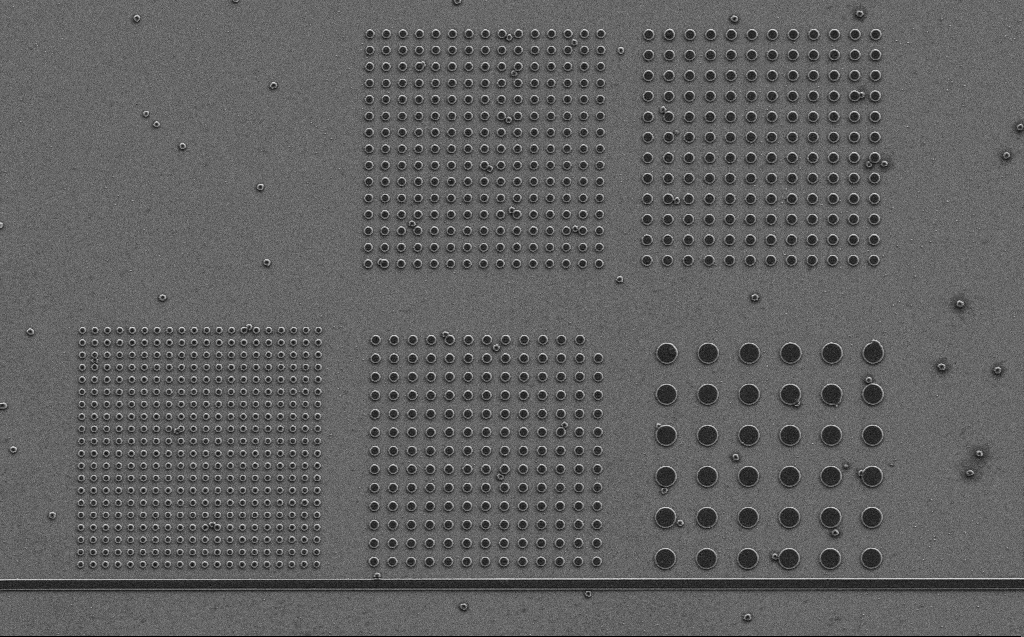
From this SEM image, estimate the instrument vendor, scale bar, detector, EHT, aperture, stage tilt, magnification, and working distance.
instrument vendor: Zeiss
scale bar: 10000 nm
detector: SE2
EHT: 3 kV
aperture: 30 µm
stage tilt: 0°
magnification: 3.82 K X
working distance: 6 mm